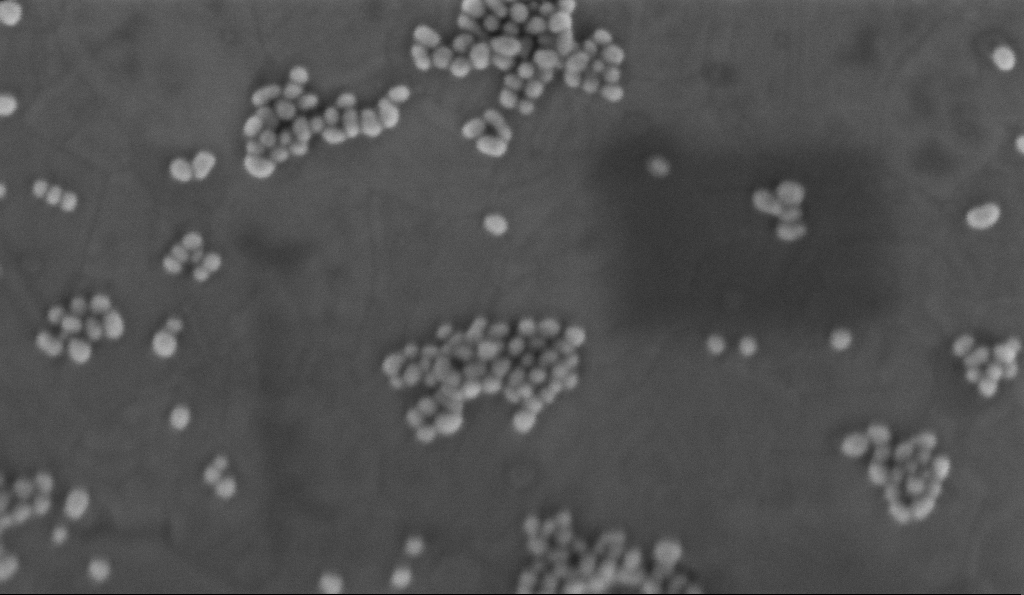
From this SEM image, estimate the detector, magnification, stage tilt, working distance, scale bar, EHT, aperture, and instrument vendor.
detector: InLens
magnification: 300 K X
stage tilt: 0°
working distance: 3.2 mm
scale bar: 100 nm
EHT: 2 kV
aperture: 30 µm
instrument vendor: Zeiss